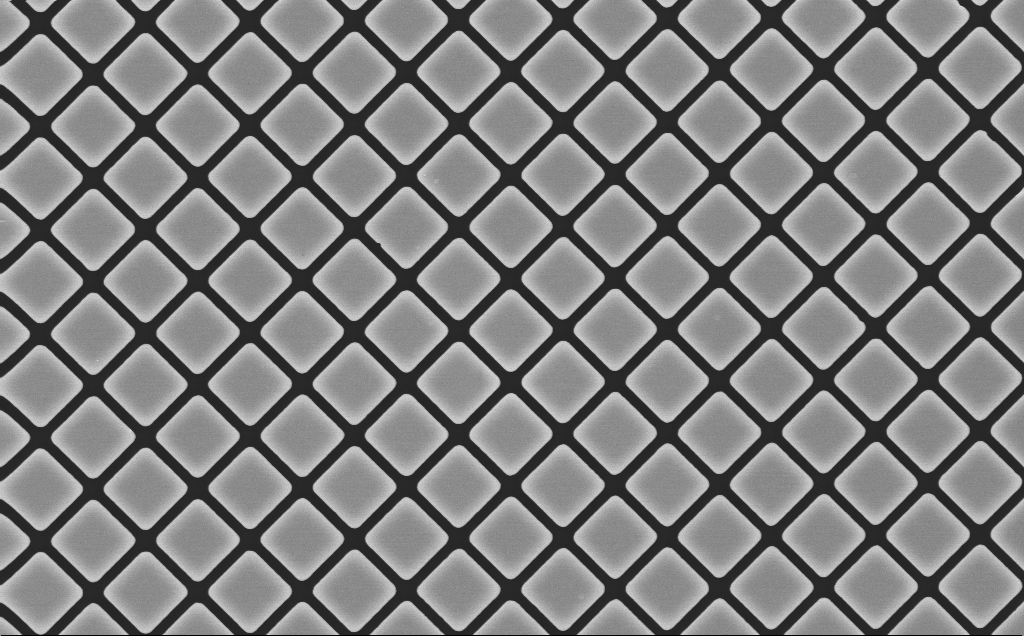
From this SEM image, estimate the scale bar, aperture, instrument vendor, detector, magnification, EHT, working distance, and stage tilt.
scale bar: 10000 nm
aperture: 30 µm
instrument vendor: Zeiss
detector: InLens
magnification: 5.46 K X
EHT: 10 kV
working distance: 9 mm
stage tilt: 0°